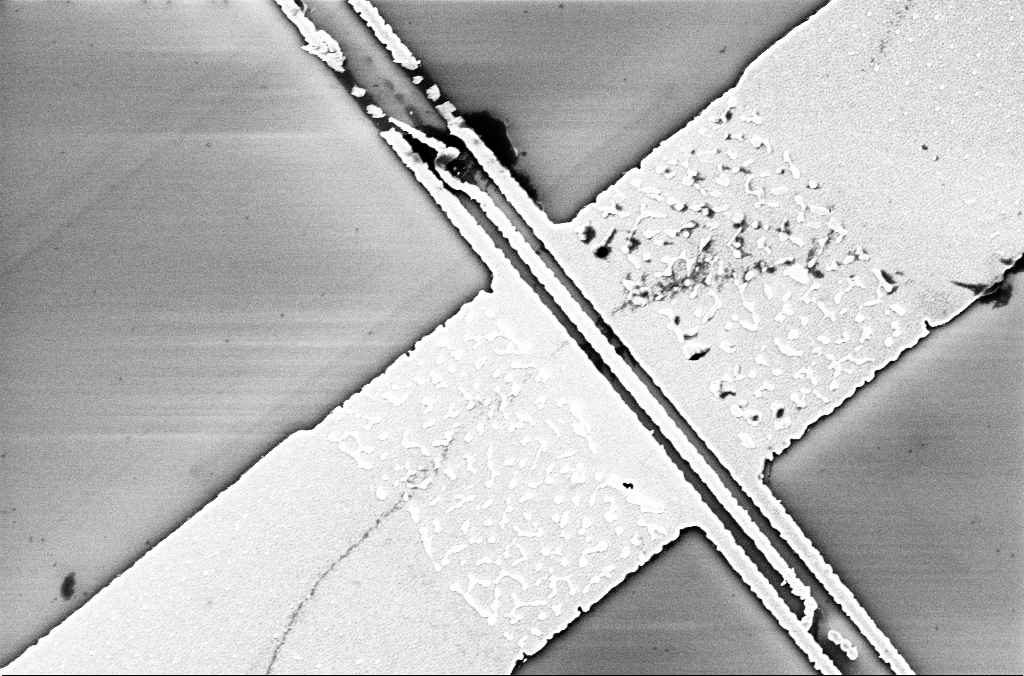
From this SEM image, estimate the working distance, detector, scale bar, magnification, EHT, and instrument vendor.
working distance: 3.3 mm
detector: InLens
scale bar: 2000 nm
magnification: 23.65 K X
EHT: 5 kV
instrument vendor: Zeiss